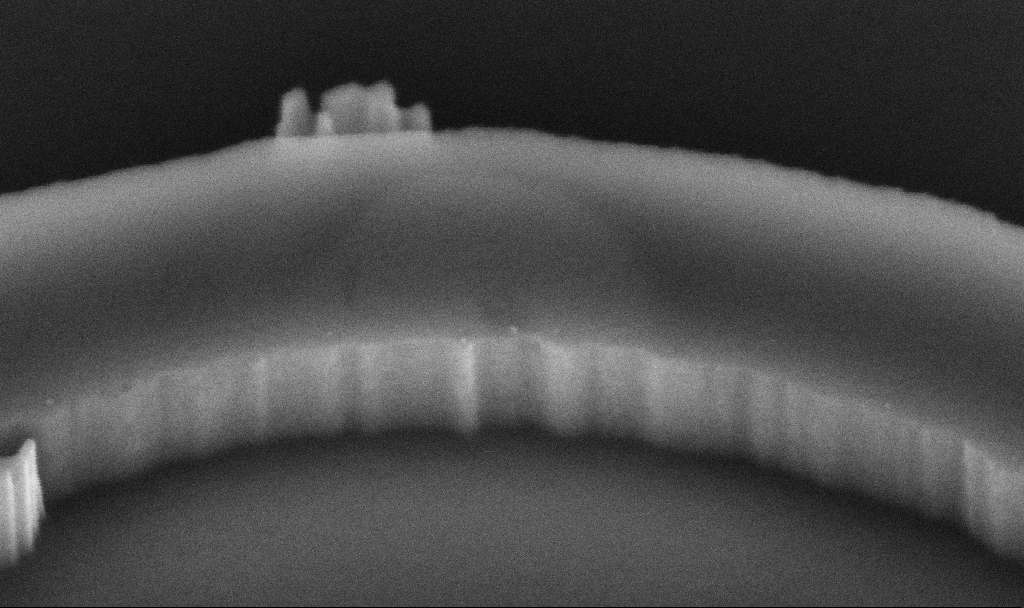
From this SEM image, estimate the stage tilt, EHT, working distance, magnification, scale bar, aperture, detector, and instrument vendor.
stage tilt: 45°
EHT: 5 kV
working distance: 10 mm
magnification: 216.81 K X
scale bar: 100 nm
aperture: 30 µm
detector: InLens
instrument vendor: Zeiss